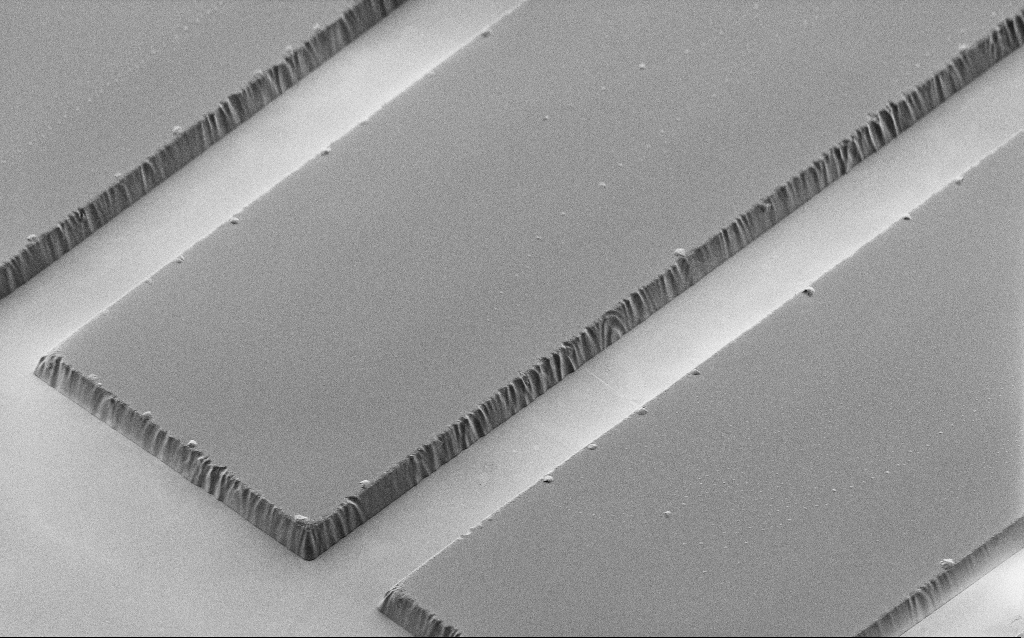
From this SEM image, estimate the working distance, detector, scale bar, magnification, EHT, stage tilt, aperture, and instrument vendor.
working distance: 7 mm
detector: SE2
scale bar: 20000 nm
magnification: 1.72 K X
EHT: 1.2 kV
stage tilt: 45°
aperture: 30 µm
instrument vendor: Zeiss